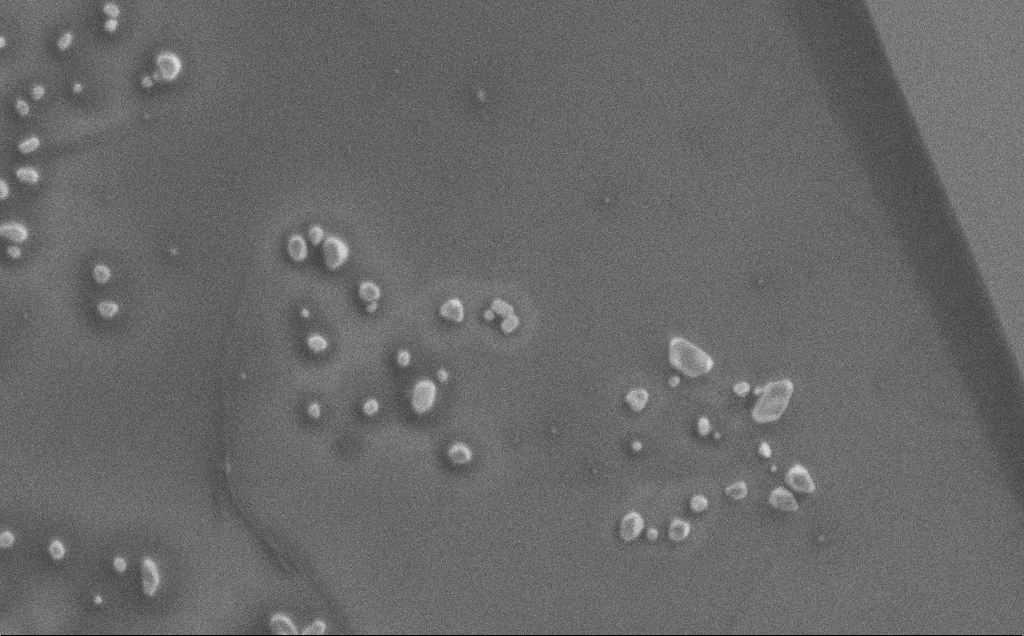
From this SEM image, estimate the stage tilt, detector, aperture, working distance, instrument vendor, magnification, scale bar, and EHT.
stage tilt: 0°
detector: SE2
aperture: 30 µm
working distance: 3 mm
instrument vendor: Zeiss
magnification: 13.15 K X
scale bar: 2000 nm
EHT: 1 kV